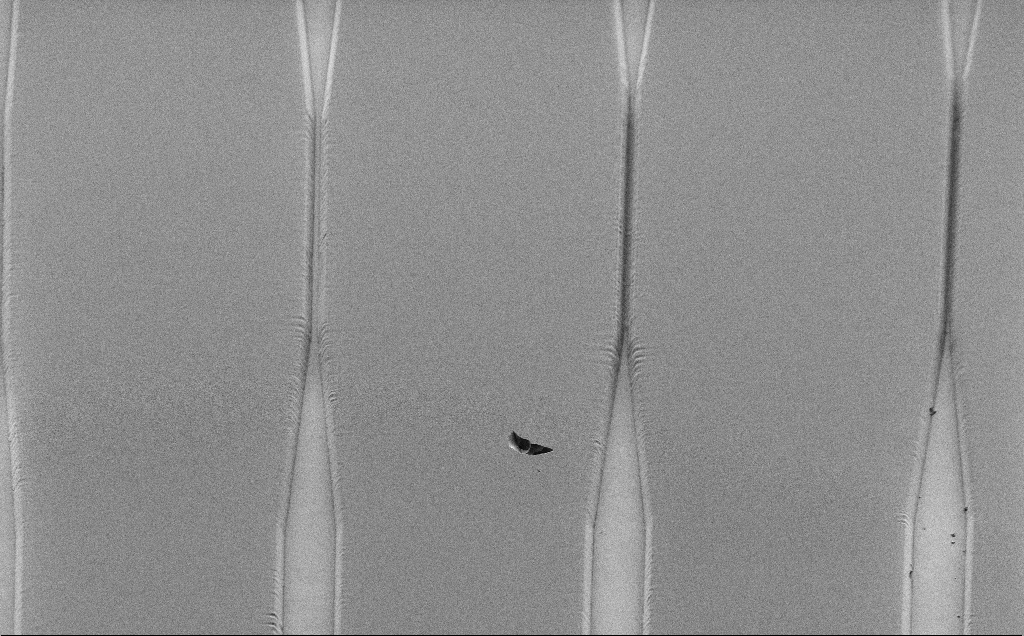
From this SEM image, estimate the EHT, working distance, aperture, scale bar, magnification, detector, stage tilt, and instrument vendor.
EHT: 1 kV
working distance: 7 mm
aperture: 30 µm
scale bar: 20000 nm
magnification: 1.09 K X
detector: SE2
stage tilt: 45°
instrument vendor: Zeiss